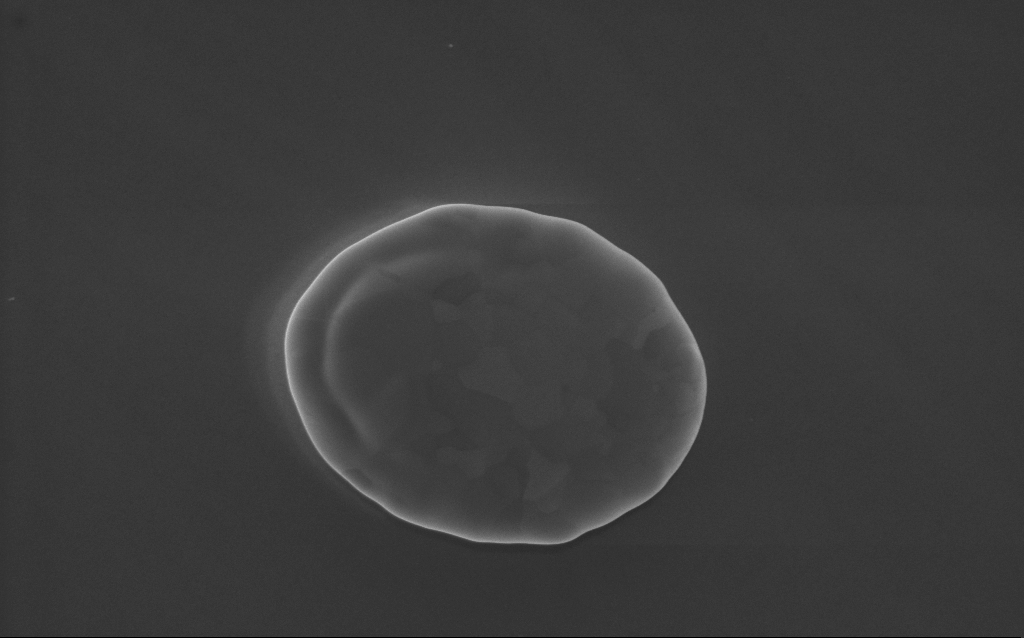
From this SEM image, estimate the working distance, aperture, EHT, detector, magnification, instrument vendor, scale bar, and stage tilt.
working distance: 3 mm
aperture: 30 µm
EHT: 5 kV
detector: InLens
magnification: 53 K X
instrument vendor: Zeiss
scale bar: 1000 nm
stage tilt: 0°